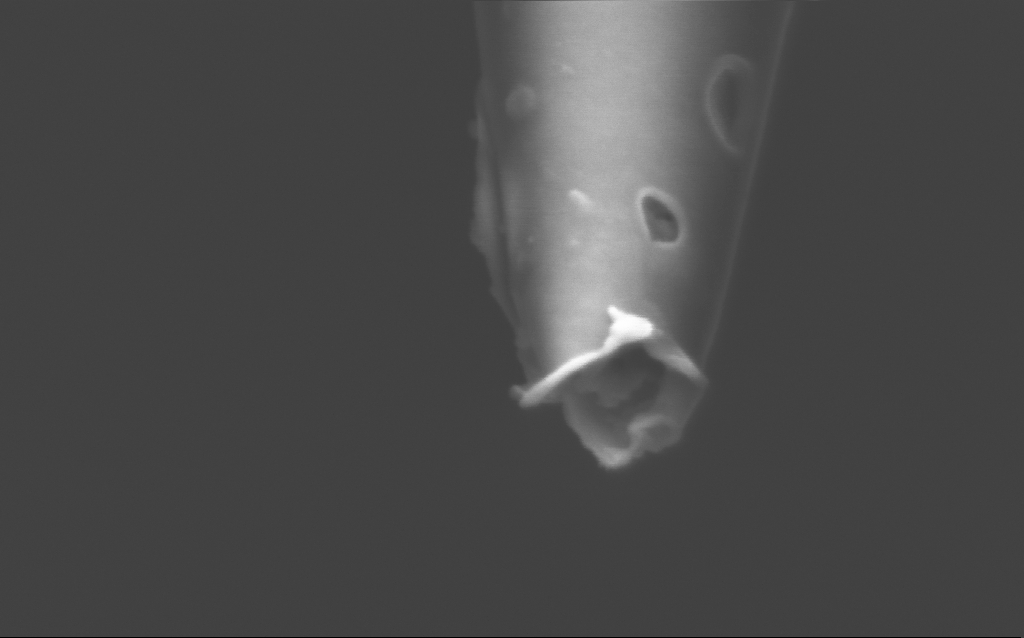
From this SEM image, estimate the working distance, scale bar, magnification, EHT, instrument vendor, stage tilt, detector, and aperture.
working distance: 6 mm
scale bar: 200 nm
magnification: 250 K X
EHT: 2 kV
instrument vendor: Zeiss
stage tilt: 45°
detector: InLens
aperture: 30 µm